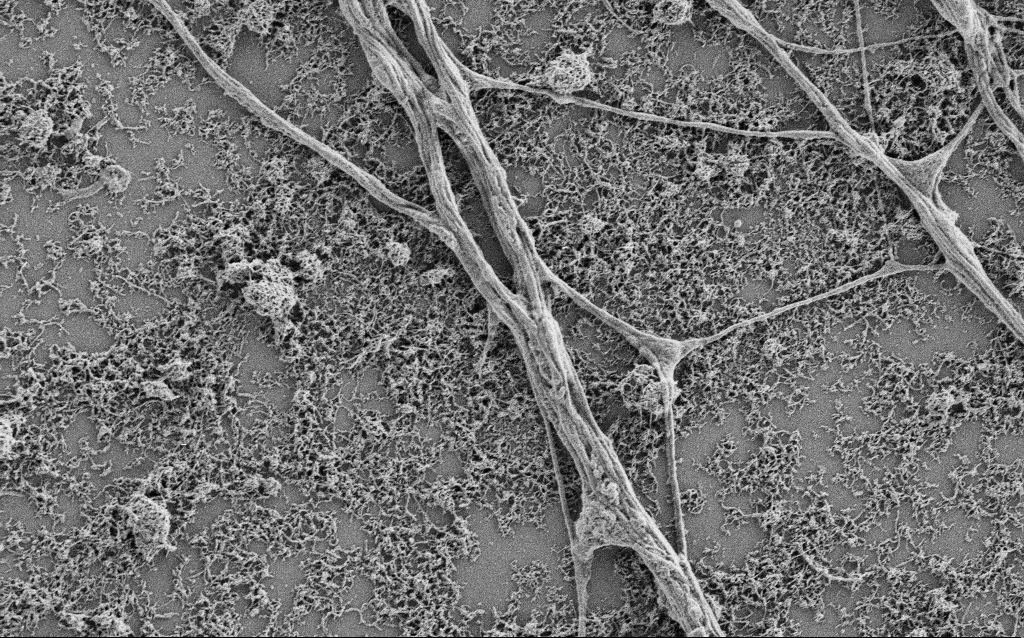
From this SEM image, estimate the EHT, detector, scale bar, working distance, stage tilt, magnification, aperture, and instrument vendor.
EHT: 1 kV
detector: SE2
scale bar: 2000 nm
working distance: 4 mm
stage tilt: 0°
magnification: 10 K X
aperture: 30 µm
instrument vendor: Zeiss